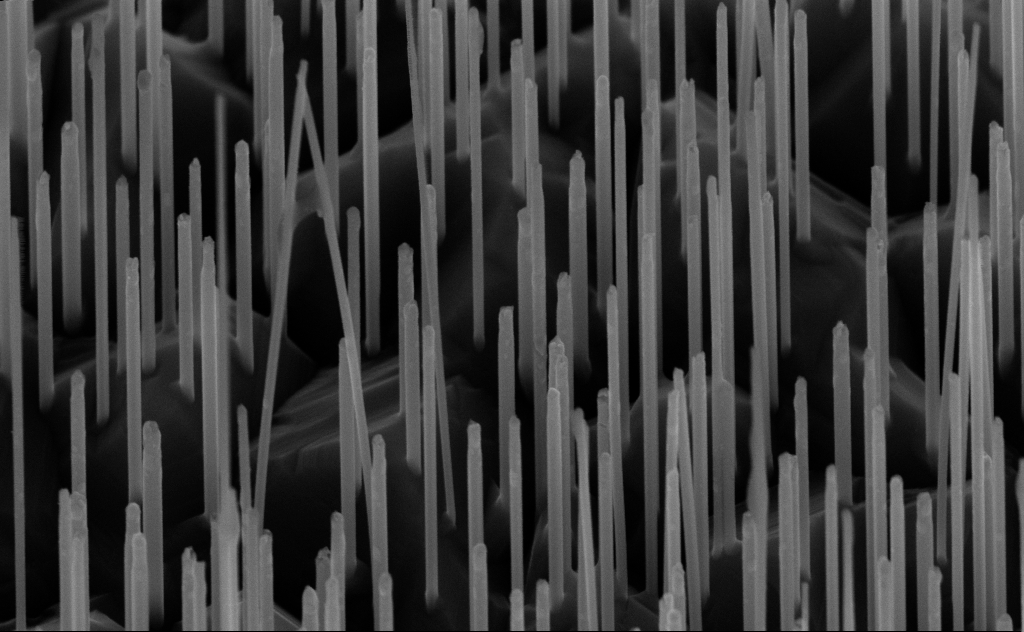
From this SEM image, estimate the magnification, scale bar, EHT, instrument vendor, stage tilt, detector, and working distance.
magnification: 80 K X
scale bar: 200 nm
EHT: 10 kV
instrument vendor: Zeiss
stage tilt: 45°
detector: InLens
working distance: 6 mm